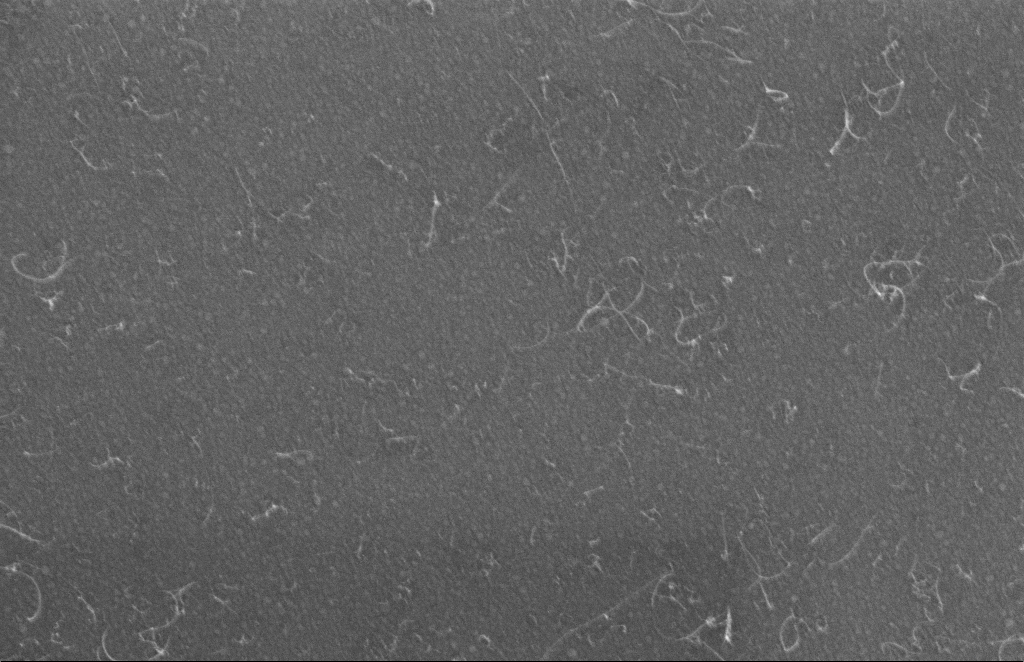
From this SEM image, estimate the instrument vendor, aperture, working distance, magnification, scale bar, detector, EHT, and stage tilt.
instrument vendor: Zeiss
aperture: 20 µm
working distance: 4 mm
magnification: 121.85 K X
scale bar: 200 nm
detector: InLens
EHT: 5 kV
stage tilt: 0°